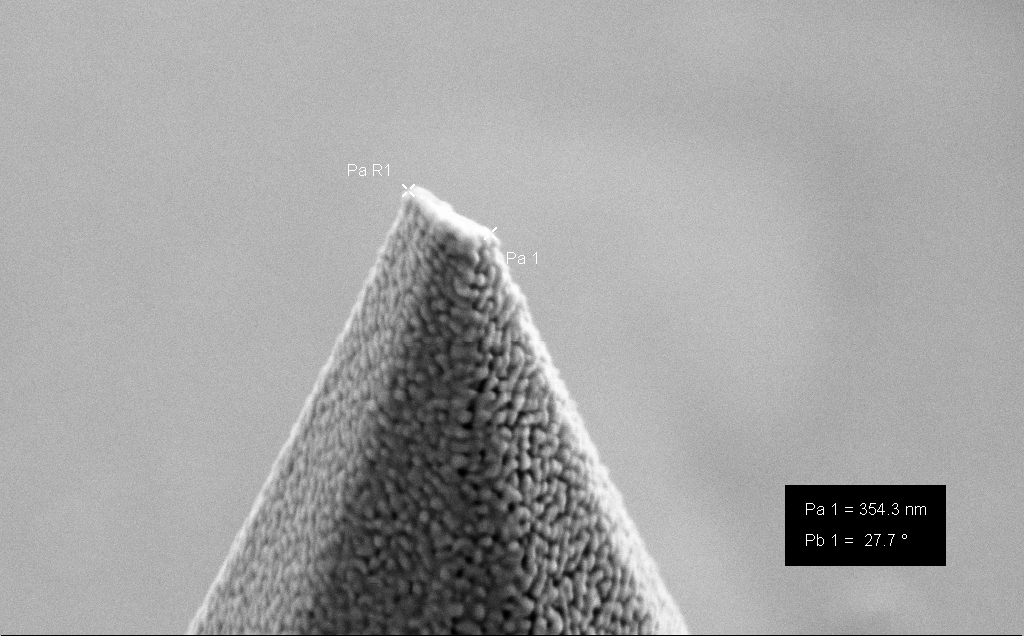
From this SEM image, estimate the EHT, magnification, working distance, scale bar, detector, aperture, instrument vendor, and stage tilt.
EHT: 5 kV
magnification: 95.95 K X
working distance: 10 mm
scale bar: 200 nm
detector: SE2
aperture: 30 µm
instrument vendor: Zeiss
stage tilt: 45.6°